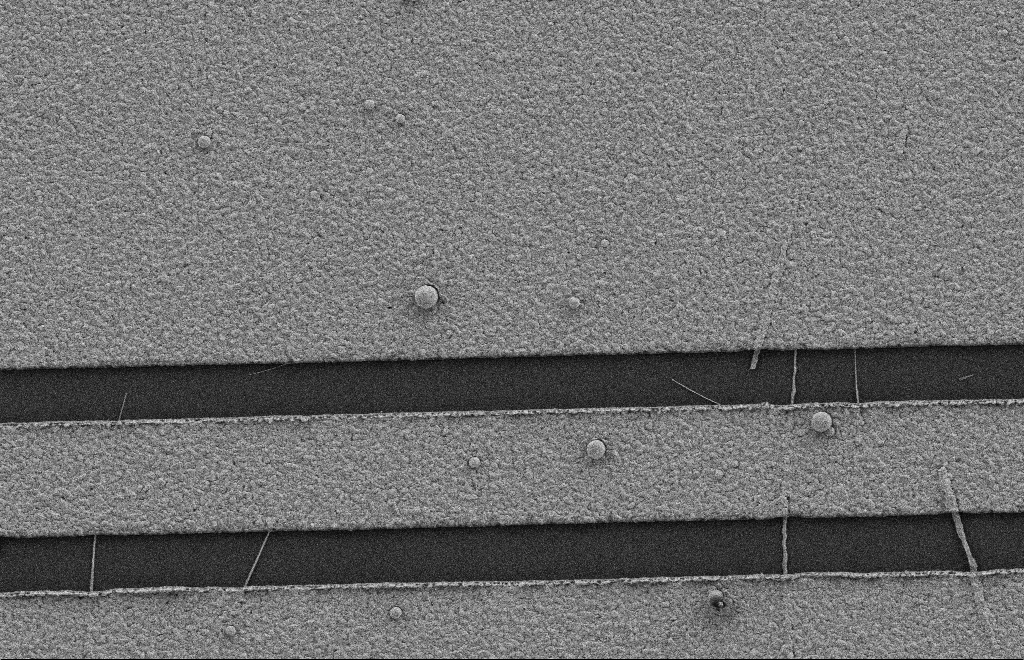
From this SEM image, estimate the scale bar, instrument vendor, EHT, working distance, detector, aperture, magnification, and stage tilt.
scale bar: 2000 nm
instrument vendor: Zeiss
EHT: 2 kV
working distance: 9 mm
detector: SE2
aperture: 20 µm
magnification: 15.61 K X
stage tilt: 0°